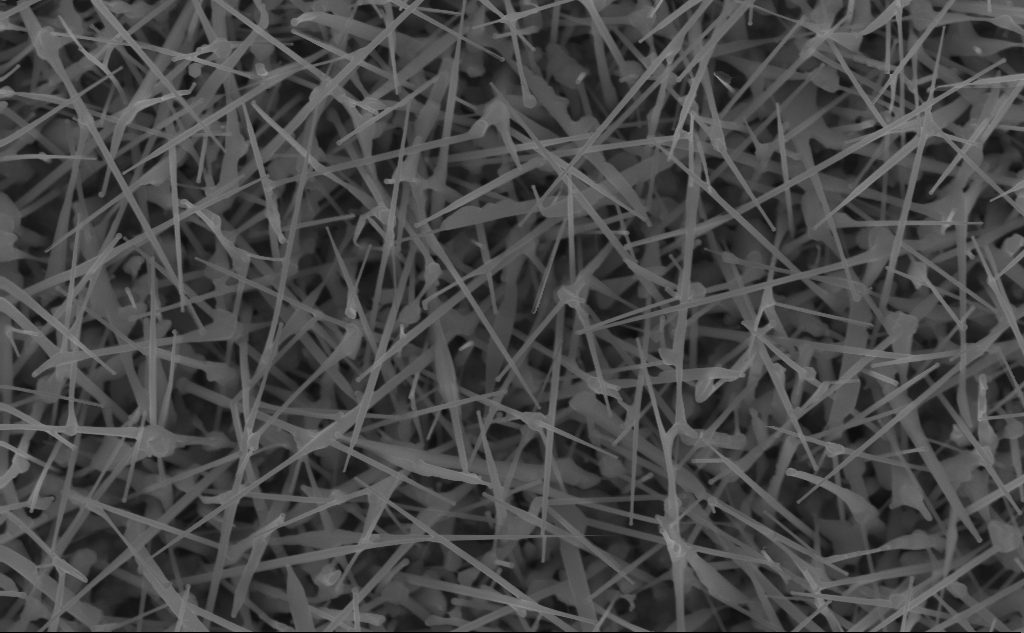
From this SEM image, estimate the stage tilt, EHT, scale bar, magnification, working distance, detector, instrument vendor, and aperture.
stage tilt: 0°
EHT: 10 kV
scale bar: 1000 nm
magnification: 39.77 K X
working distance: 5 mm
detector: InLens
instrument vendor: Zeiss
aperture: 30 µm